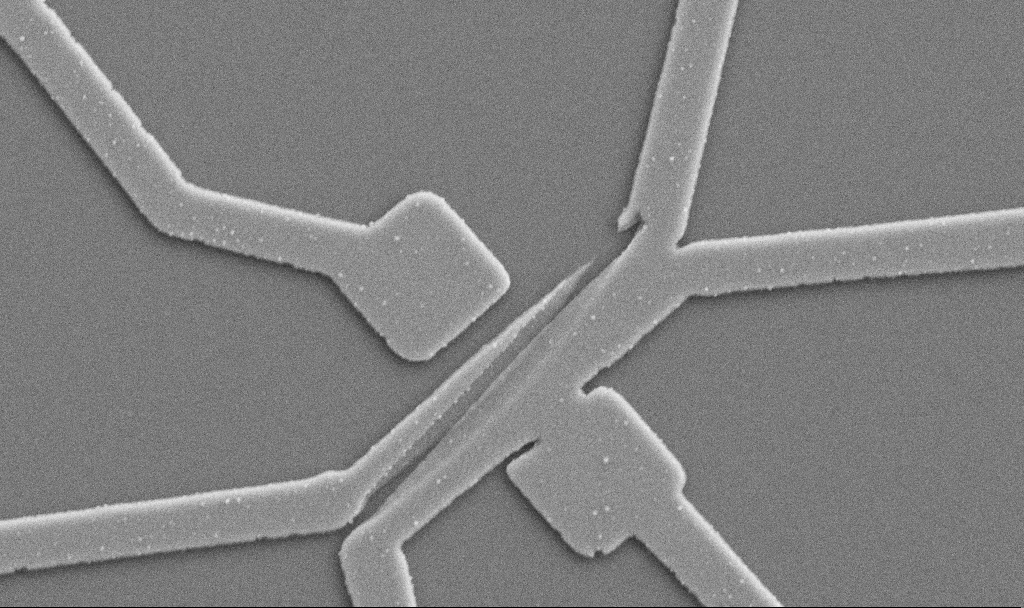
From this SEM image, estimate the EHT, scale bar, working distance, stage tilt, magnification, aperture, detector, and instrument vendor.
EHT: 5 kV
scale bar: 1000 nm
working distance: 10.7 mm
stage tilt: -0°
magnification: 20 K X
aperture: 30 µm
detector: SE2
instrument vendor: Zeiss